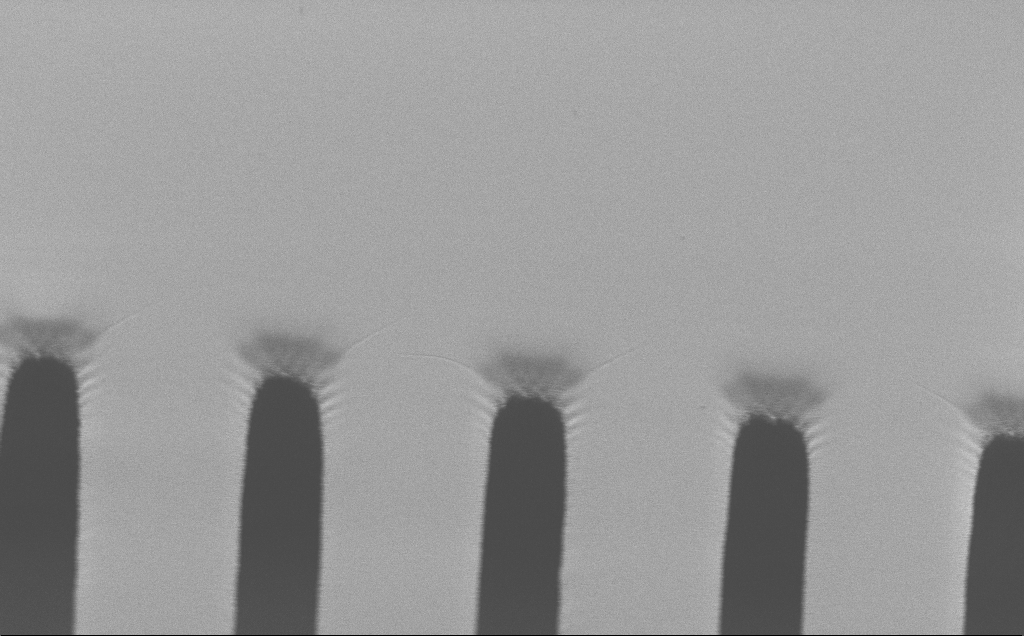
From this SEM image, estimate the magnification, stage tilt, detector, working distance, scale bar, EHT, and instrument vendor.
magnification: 2.79 K X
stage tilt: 45°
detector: SE2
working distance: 7 mm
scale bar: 10000 nm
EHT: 1.2 kV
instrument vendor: Zeiss